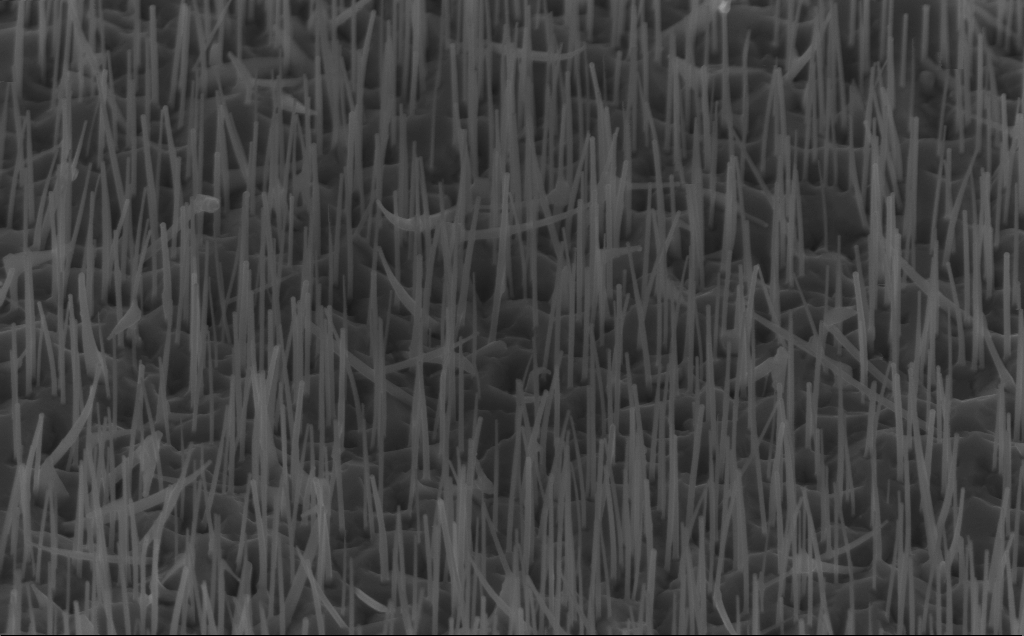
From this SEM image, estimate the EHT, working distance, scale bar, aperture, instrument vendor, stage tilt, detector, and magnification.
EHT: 10 kV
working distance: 5 mm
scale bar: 1000 nm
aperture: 30 µm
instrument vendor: Zeiss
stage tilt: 45°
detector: InLens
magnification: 40 K X